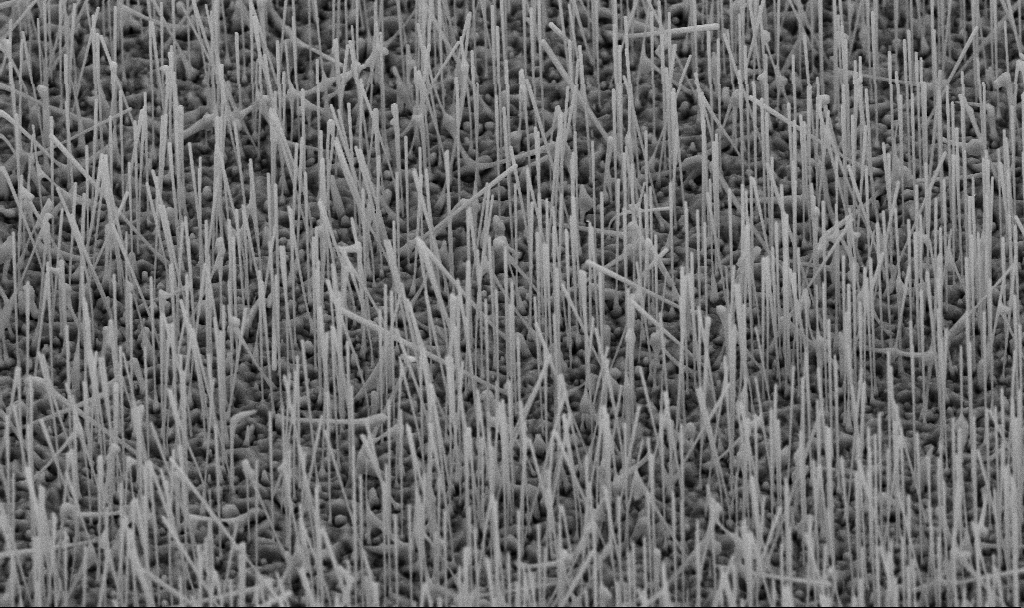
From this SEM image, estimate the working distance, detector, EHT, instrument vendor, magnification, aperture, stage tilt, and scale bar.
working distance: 7.2 mm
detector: SE2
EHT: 10 kV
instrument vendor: Zeiss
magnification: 10 K X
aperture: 30 µm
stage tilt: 45°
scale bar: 2000 nm